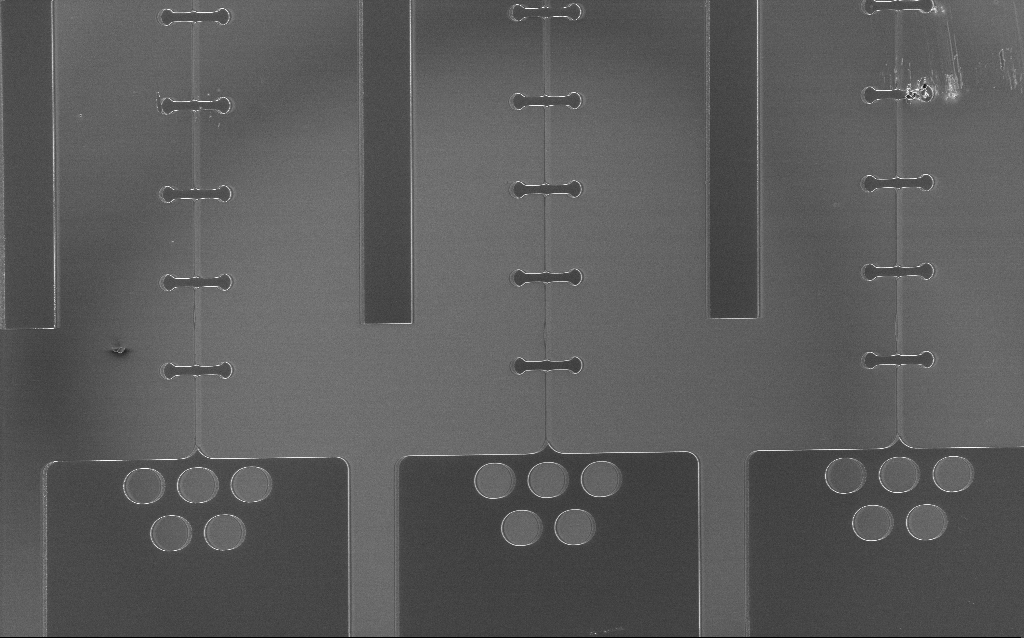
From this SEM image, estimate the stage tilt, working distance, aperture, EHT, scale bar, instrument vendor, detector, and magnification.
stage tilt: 0°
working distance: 5 mm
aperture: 30 µm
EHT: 3 kV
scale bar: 200000 nm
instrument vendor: Zeiss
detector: InLens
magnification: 0.327 K X